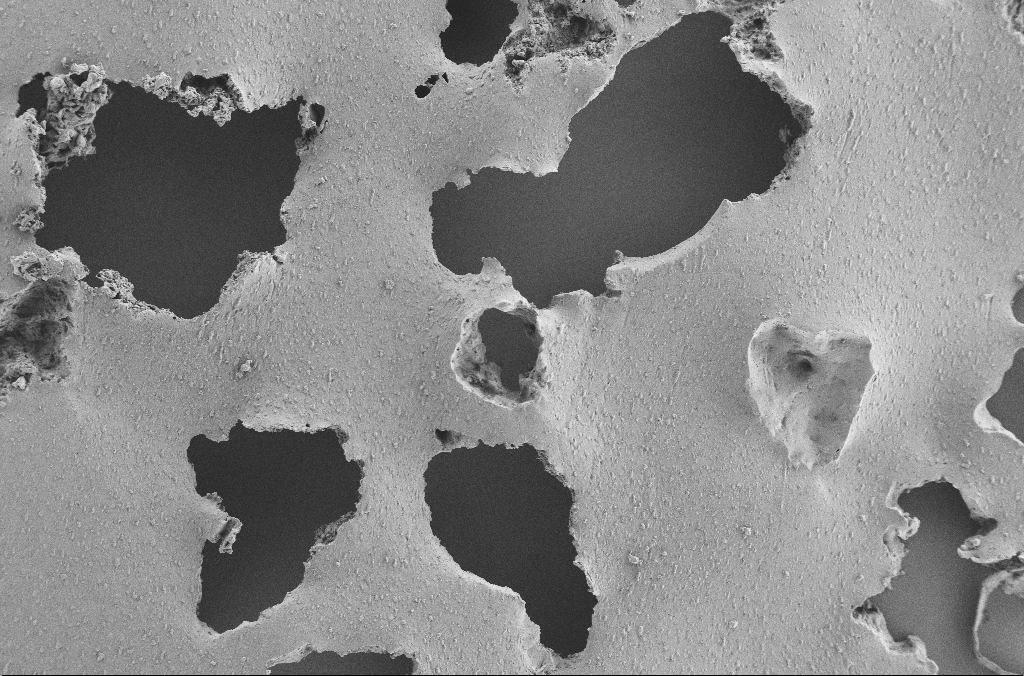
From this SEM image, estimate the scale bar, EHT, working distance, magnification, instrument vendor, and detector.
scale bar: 100000 nm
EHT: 2 kV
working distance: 3.6 mm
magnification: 0.25 K X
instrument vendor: Zeiss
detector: SE2